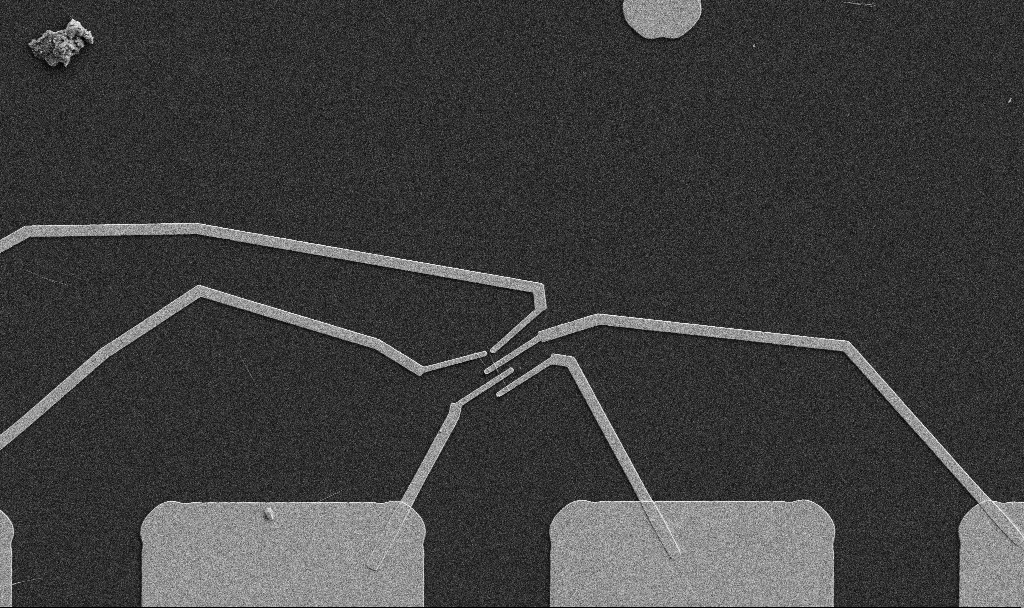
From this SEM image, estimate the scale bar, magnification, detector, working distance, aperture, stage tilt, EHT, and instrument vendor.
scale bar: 10000 nm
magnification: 5 K X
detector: SE2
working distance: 10.7 mm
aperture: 30 µm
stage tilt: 0°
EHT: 5 kV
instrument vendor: Zeiss